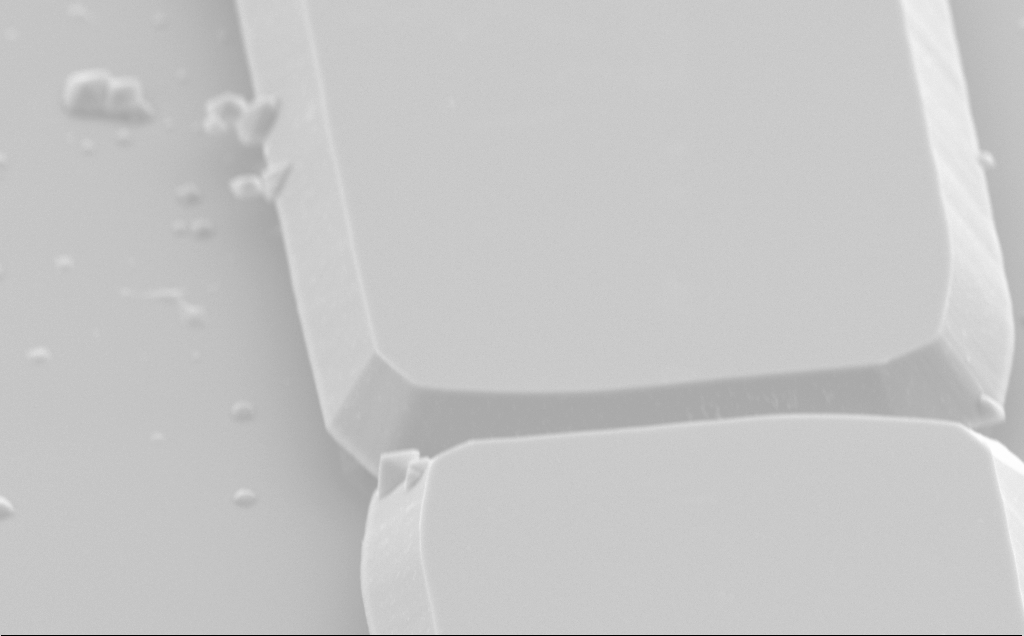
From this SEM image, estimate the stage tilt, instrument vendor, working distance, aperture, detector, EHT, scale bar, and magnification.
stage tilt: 50°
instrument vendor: Zeiss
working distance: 10 mm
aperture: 30 µm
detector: SE2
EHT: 5 kV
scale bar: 2000 nm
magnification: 10.96 K X